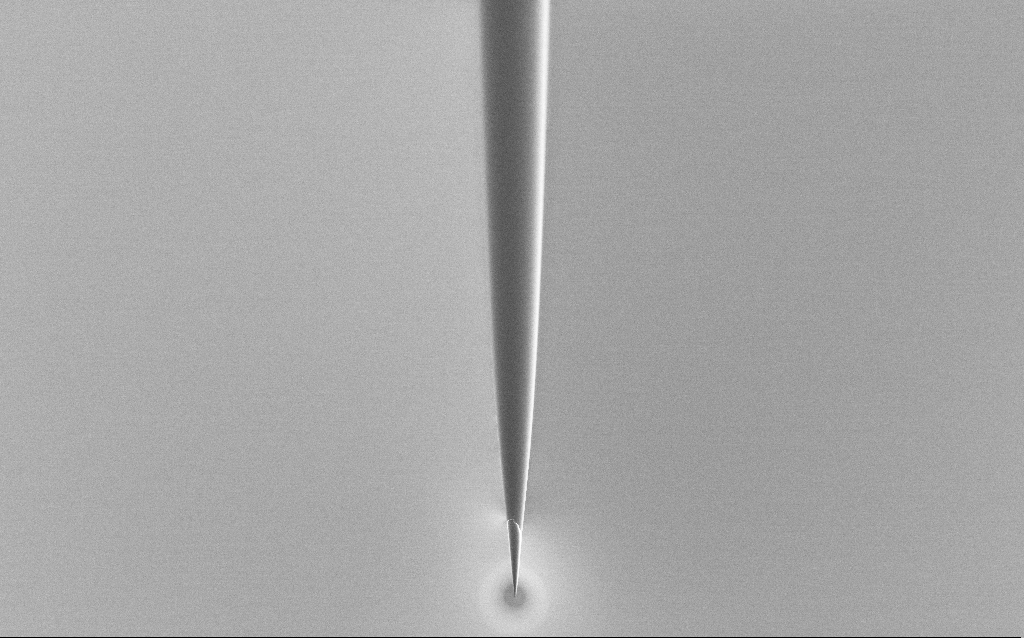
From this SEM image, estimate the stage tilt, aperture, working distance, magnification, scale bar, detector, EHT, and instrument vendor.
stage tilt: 45°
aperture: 30 µm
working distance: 6 mm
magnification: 1 K X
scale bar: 20000 nm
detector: SE2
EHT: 1 kV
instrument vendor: Zeiss